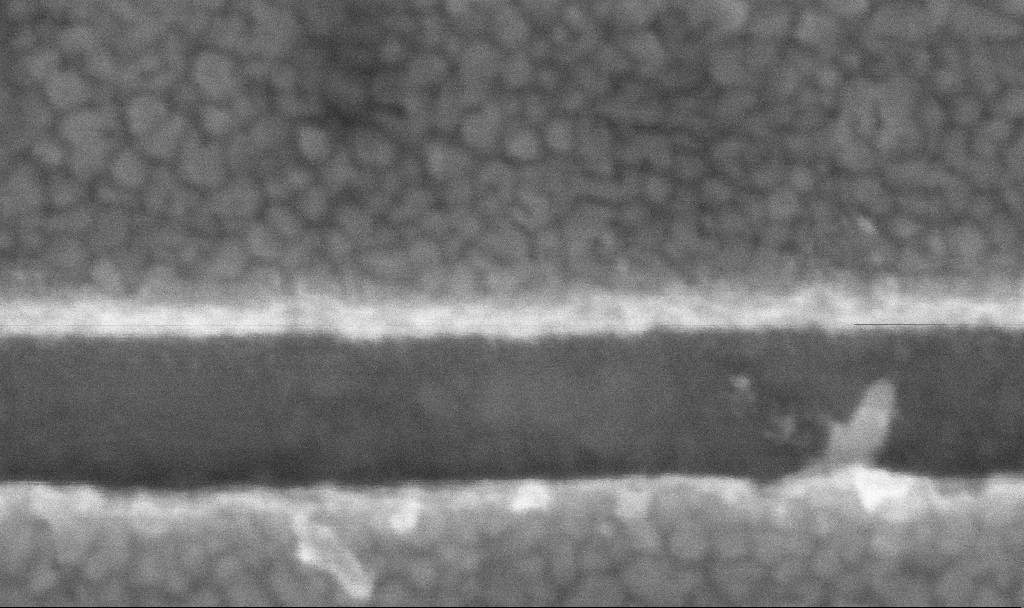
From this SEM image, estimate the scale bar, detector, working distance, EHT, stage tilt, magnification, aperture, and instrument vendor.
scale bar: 100 nm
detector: InLens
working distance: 3.8 mm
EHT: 5 kV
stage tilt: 0°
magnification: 531.29 K X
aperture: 60 µm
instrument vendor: Zeiss